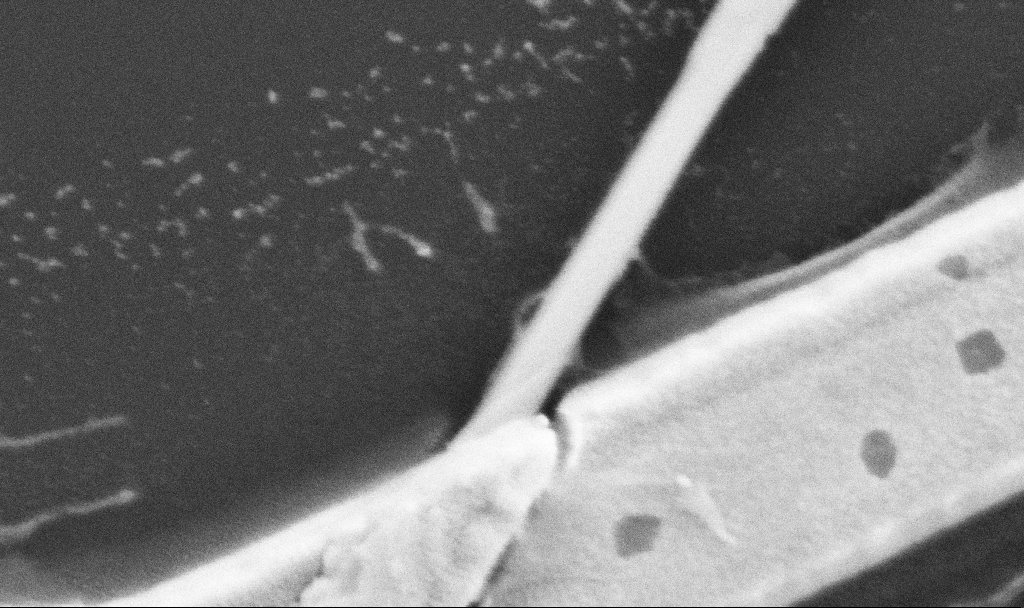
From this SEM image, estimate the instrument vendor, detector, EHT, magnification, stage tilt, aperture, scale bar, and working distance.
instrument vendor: Zeiss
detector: SE2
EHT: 5 kV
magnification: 150 K X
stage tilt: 0°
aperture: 30 µm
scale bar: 100 nm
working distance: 10.7 mm